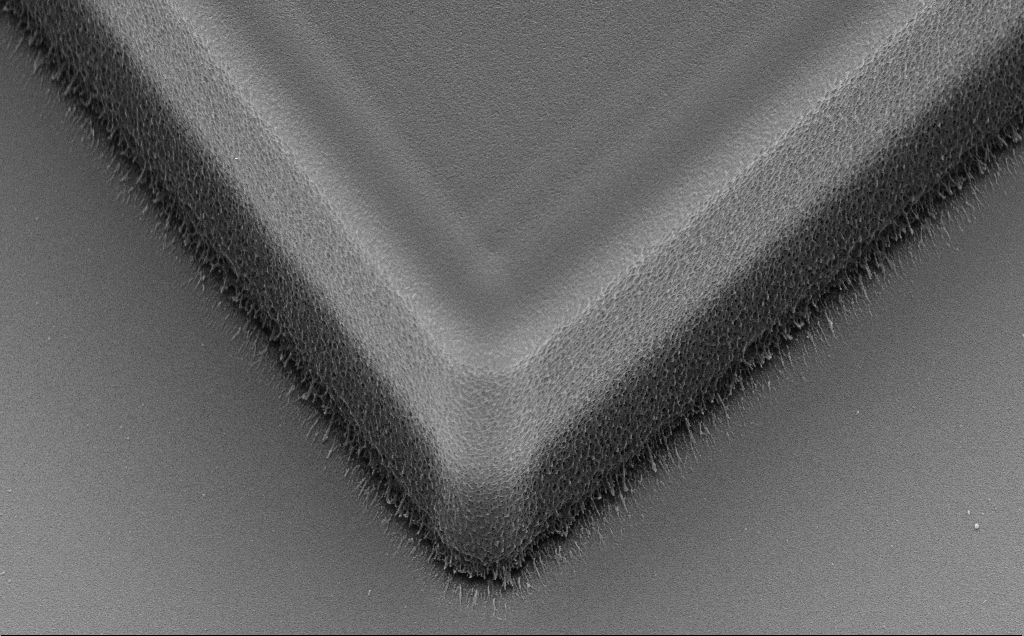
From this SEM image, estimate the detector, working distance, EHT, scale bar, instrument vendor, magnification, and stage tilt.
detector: SE2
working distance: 11 mm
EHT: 10 kV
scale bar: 2000 nm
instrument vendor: Zeiss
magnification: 9.74 K X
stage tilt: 35.3°